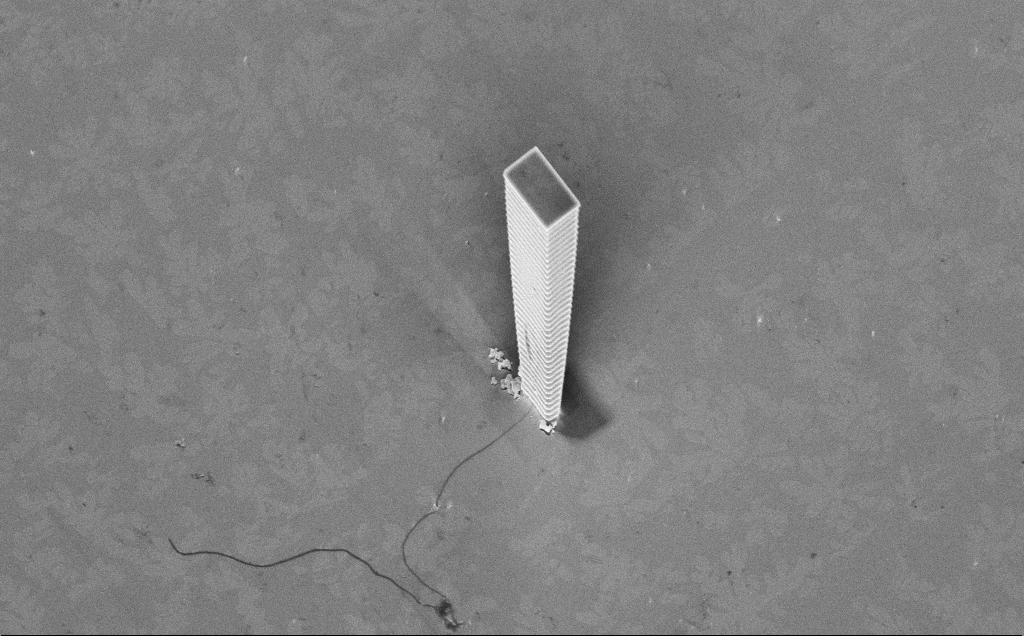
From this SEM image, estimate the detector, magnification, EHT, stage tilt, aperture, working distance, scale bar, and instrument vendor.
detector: InLens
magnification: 5.58 K X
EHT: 5 kV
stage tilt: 45°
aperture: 30 µm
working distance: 14 mm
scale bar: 10000 nm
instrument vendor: Zeiss